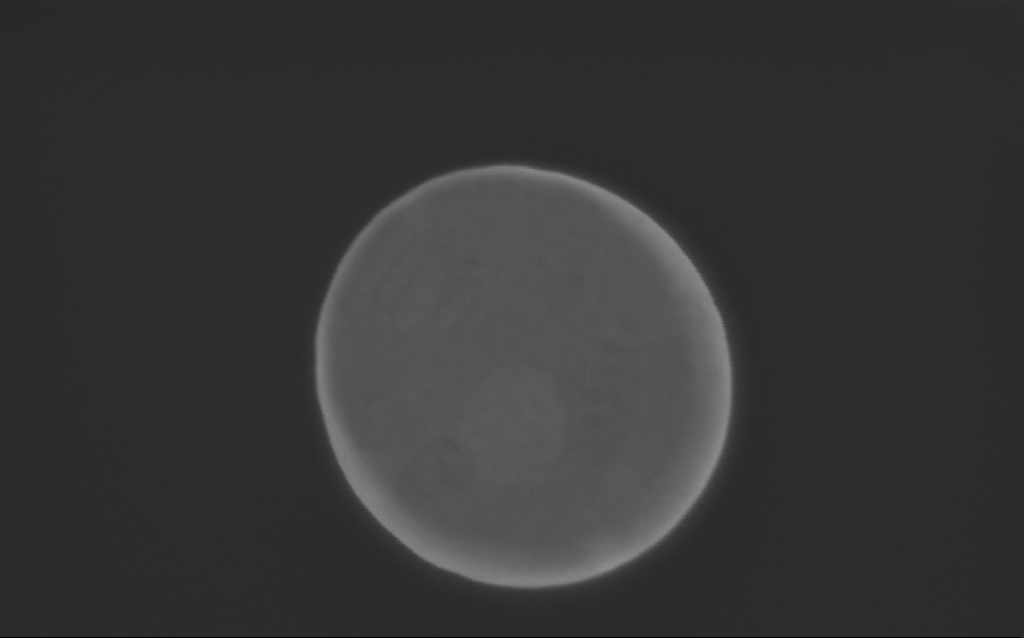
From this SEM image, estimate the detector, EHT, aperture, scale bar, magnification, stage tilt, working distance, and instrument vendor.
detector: InLens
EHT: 3 kV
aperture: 30 µm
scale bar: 200 nm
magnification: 209.14 K X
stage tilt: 0°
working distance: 3 mm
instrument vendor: Zeiss